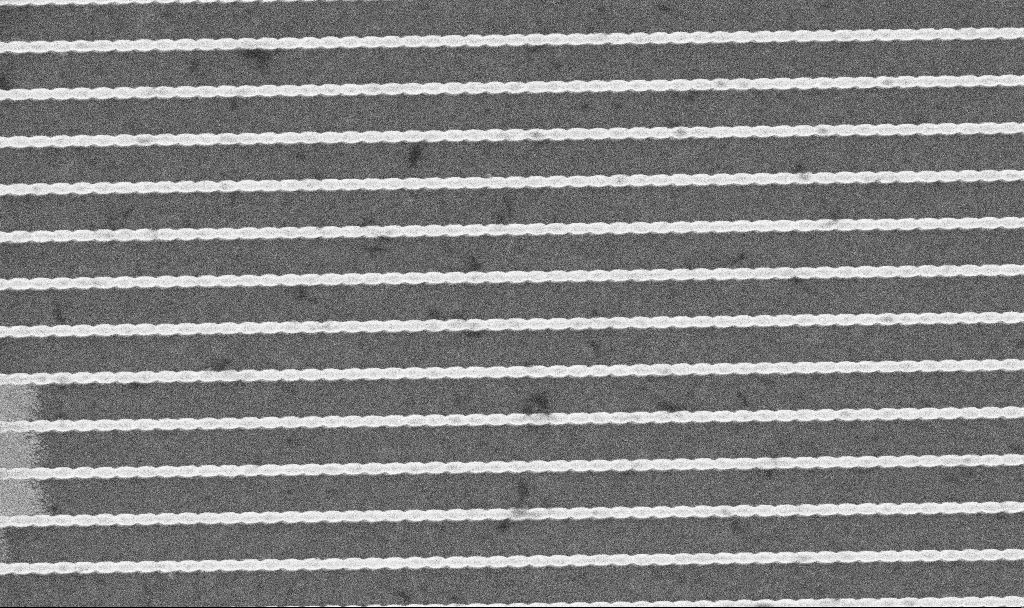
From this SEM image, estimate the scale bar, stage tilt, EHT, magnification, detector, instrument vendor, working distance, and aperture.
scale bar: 1000 nm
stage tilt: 0°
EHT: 5 kV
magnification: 58.33 K X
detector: InLens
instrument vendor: Zeiss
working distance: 4.2 mm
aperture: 30 µm